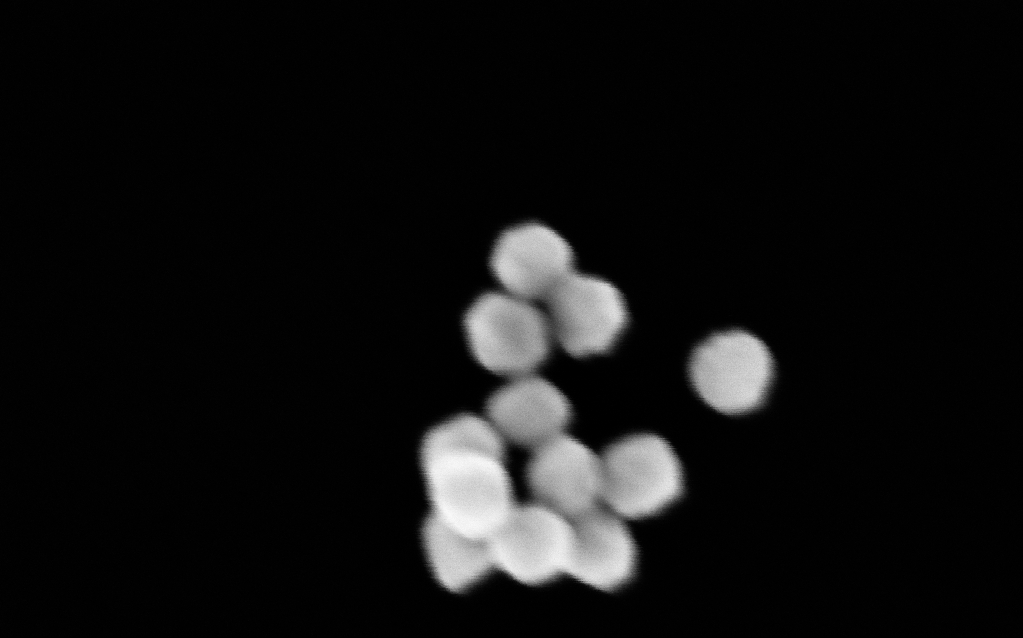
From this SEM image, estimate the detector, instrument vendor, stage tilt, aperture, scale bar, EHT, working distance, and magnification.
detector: InLens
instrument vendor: Zeiss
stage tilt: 0°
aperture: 30 µm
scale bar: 100 nm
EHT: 5 kV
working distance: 3.1 mm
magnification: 500 K X